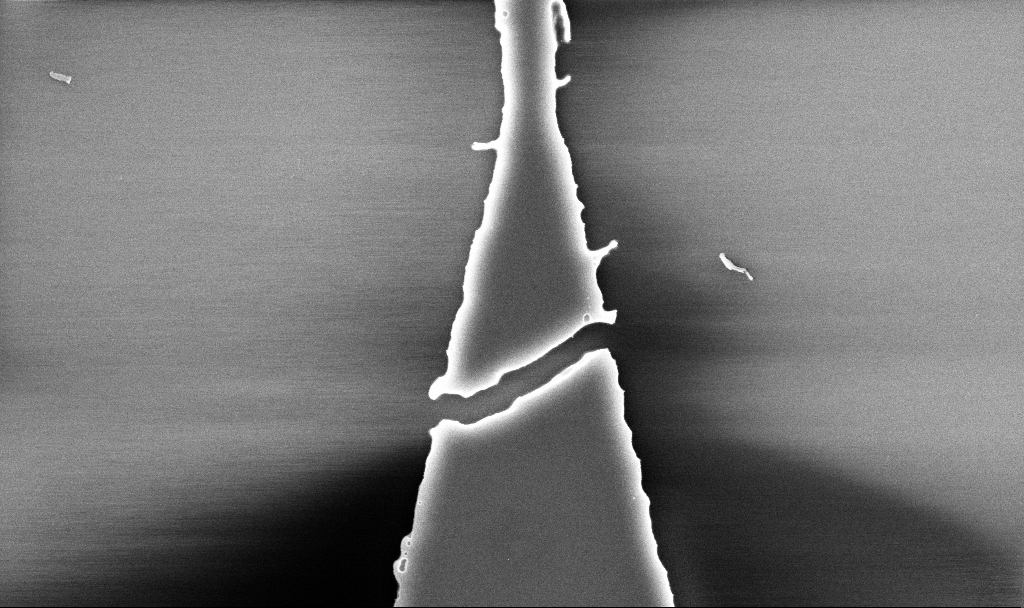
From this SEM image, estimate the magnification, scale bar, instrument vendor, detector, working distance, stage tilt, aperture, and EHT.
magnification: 37.51 K X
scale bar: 1000 nm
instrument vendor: Zeiss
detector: InLens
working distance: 5.2 mm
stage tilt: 0°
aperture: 30 µm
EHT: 5 kV